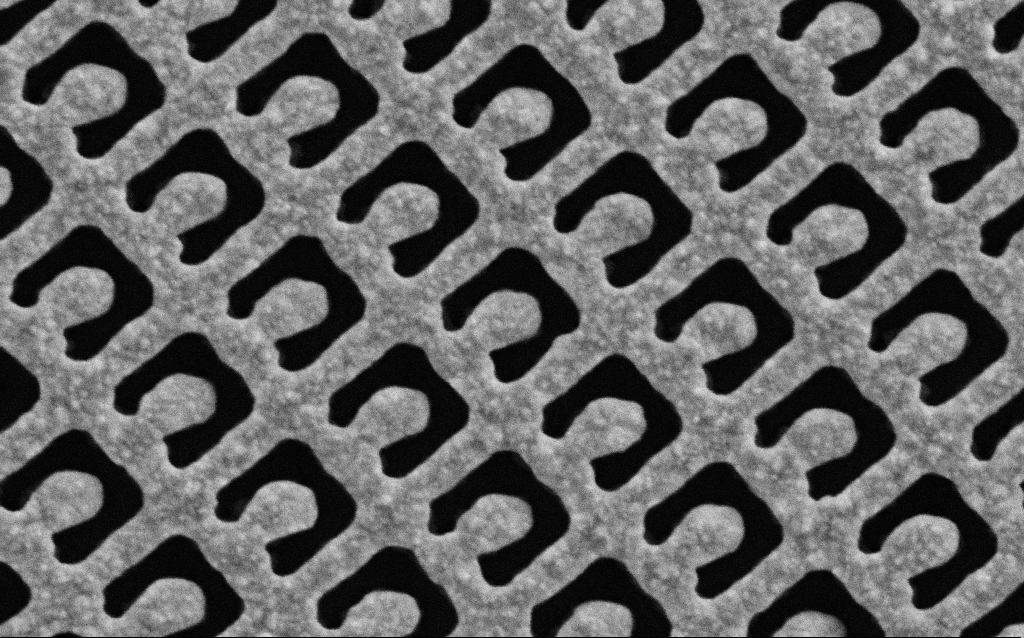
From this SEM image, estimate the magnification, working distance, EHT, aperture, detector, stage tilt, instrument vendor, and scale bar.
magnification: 120.77 K X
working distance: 6.1 mm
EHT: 5 kV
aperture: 30 µm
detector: SE2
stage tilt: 0°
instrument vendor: Zeiss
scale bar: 200 nm